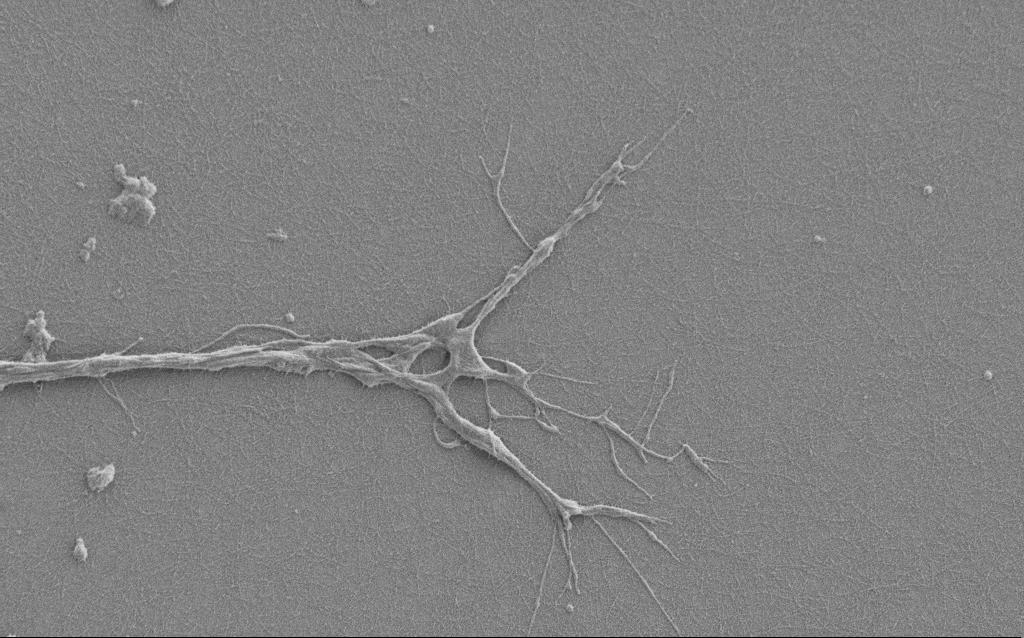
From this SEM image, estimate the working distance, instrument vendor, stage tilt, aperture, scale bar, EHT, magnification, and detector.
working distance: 6 mm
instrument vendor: Zeiss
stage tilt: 0°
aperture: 30 µm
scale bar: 10000 nm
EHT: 1 kV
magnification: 6.5 K X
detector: SE2